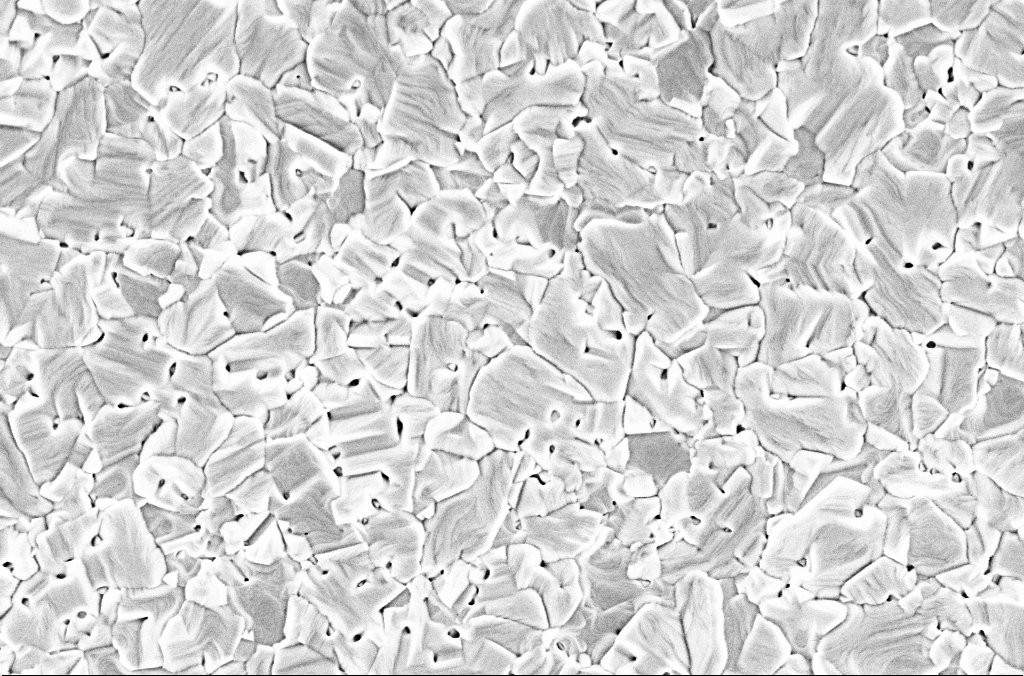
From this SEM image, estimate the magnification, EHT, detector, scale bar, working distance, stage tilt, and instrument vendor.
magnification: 40 K X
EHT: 2 kV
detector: InLens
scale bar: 1000 nm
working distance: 3 mm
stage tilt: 0°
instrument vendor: Zeiss